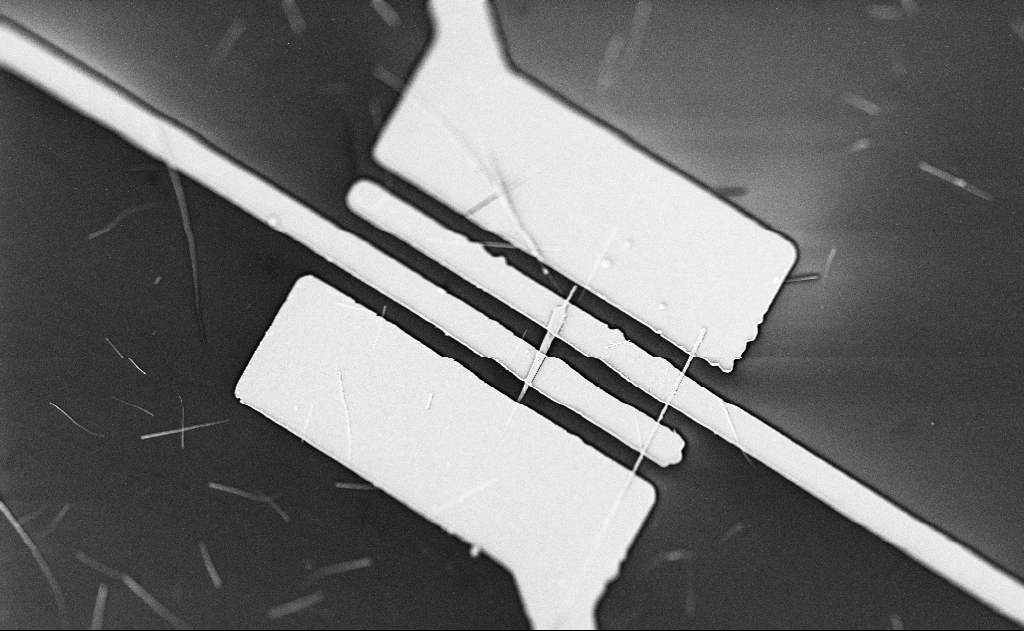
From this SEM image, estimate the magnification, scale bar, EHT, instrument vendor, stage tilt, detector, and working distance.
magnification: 5 K X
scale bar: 10000 nm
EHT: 5 kV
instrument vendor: Zeiss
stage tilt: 0°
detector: SE2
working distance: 20 mm